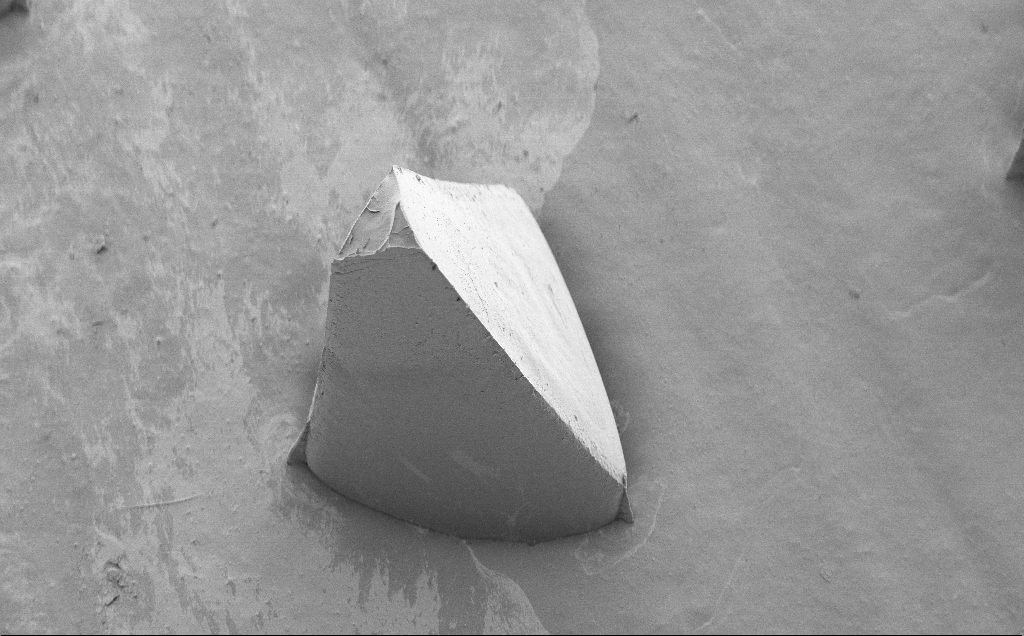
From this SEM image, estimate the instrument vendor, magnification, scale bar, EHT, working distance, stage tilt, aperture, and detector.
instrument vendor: Zeiss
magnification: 0.187 K X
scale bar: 200000 nm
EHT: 5 kV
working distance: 8 mm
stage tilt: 40°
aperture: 30 µm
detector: SE2